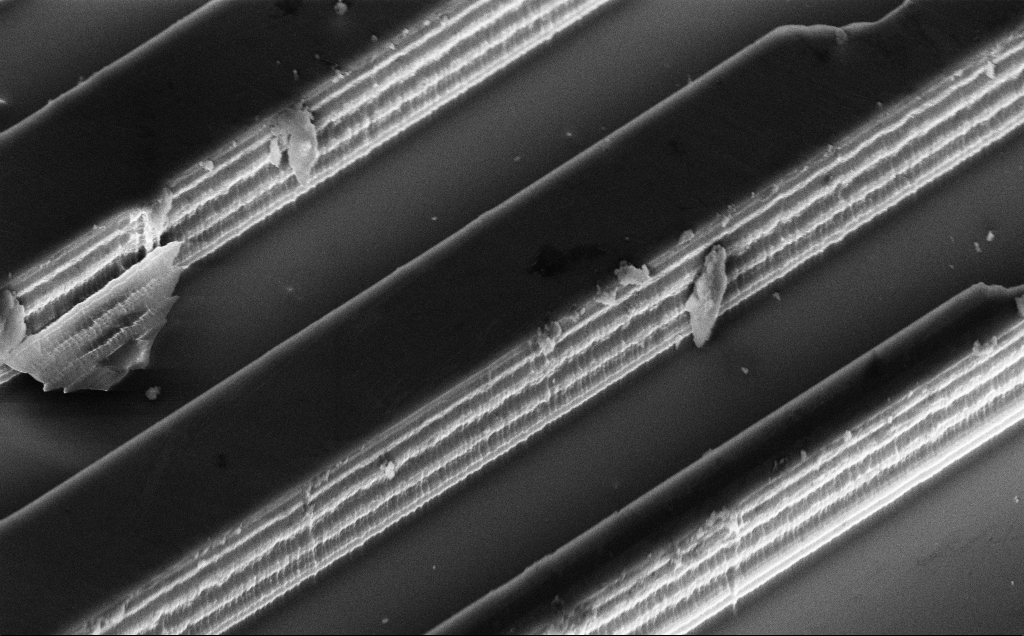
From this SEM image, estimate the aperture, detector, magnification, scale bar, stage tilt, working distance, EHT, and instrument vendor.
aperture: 30 µm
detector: InLens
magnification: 6.02 K X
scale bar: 10000 nm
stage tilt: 50°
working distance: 10 mm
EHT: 10 kV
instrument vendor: Zeiss